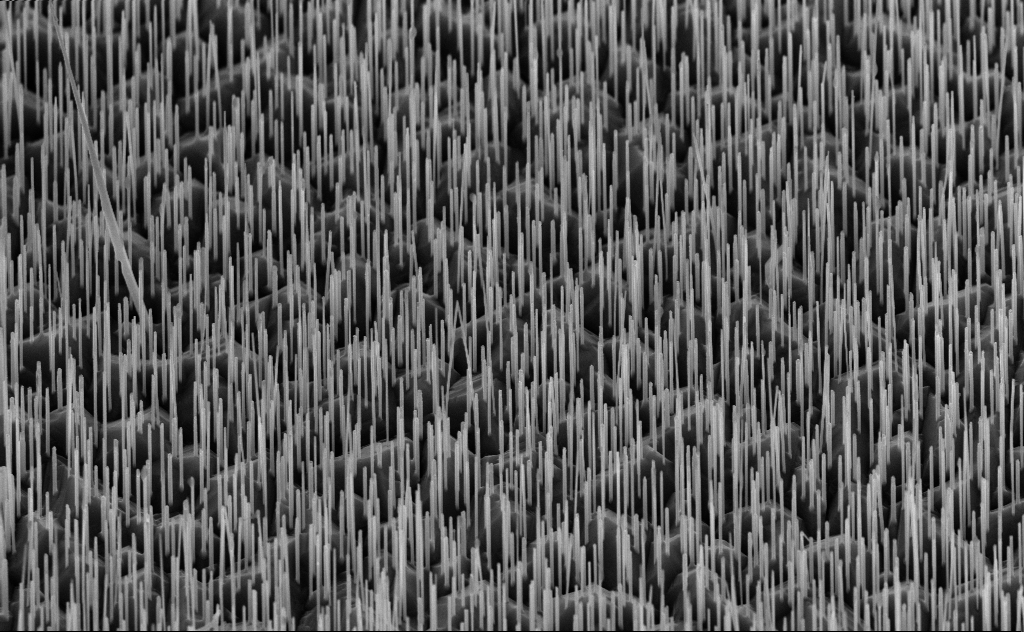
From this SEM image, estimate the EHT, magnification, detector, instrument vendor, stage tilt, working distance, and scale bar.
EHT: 10 kV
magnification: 20 K X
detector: InLens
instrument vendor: Zeiss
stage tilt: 45°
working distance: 6 mm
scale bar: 2000 nm